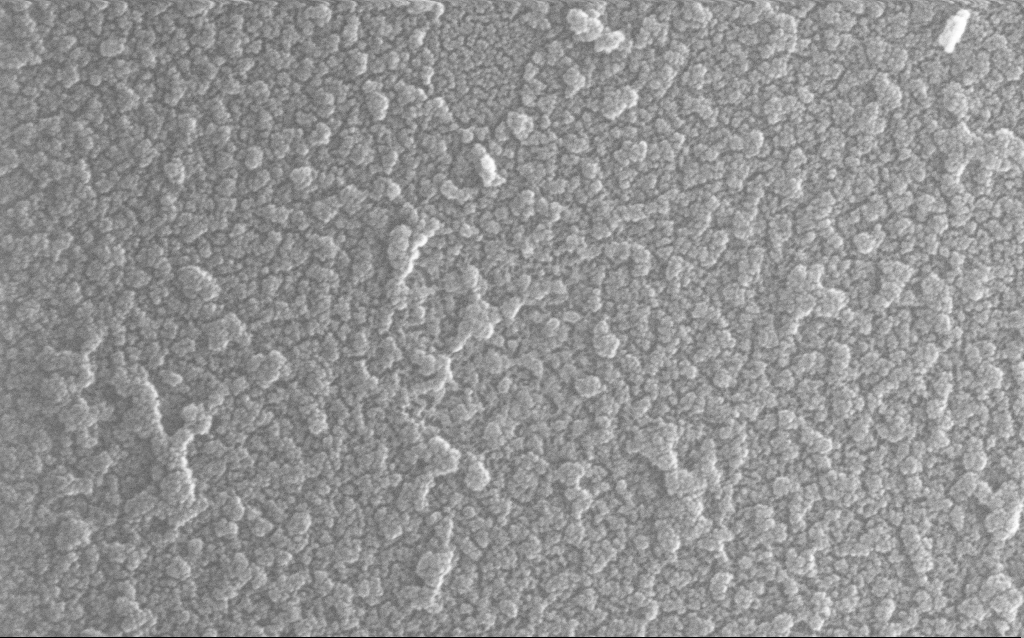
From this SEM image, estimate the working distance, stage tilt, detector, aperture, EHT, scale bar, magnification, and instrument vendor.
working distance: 1.6 mm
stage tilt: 0°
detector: InLens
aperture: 30 µm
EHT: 20 kV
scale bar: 100 nm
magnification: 300 K X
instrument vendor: Zeiss